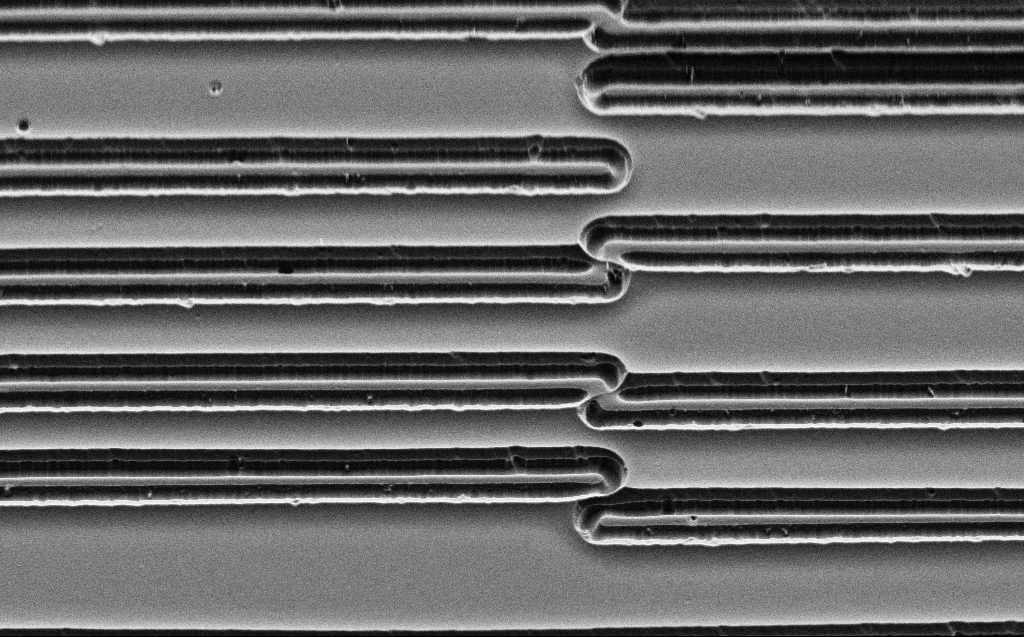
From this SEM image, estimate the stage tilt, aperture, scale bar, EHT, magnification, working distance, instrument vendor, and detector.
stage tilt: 45°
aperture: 30 µm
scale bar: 10000 nm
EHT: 3 kV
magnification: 4.38 K X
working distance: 6 mm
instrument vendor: Zeiss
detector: SE2